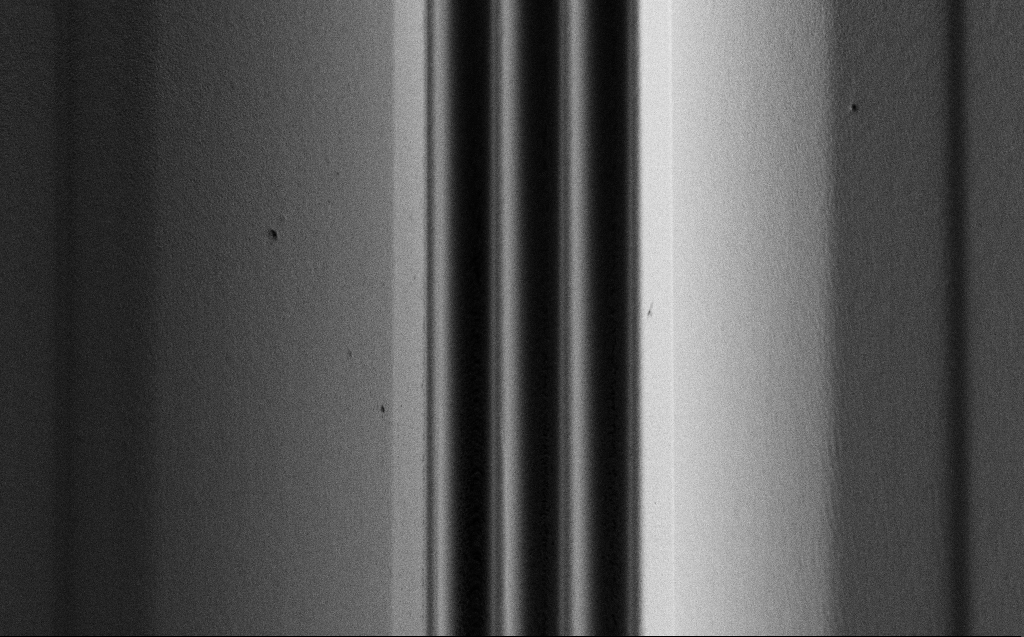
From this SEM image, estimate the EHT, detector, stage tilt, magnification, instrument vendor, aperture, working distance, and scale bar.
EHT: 0.9 kV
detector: SE2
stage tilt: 45°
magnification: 2.24 K X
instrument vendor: Zeiss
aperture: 30 µm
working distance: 6 mm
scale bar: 20000 nm